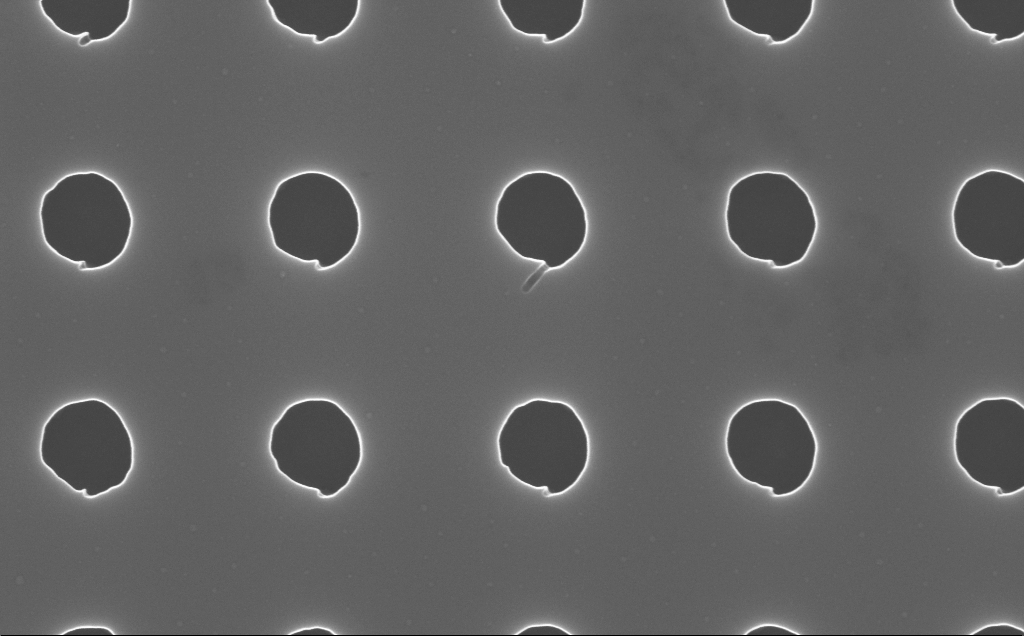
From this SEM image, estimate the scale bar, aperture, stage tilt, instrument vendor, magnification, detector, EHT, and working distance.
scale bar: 200 nm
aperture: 30 µm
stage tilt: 0°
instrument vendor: Zeiss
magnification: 80 K X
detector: InLens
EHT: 10 kV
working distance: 4 mm